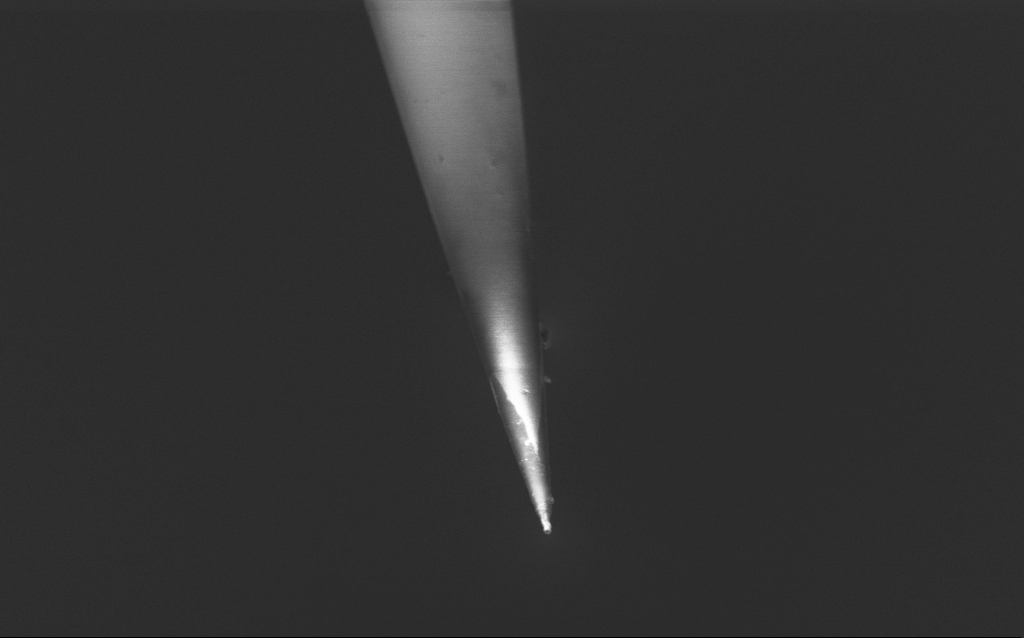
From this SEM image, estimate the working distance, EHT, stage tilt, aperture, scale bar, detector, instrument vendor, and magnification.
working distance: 6 mm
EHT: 1.5 kV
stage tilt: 45°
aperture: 30 µm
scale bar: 2000 nm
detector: InLens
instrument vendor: Zeiss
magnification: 25 K X